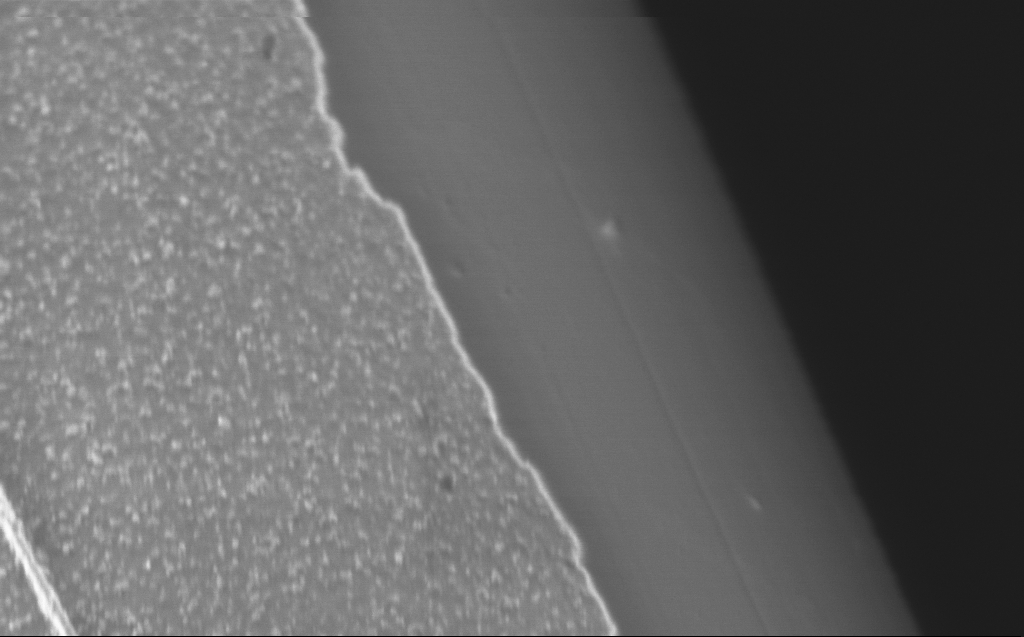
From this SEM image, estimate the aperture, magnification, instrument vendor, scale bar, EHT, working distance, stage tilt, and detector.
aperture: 30 µm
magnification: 75 K X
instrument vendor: Zeiss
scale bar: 200 nm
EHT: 0.8 kV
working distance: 4 mm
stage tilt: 45°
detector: InLens